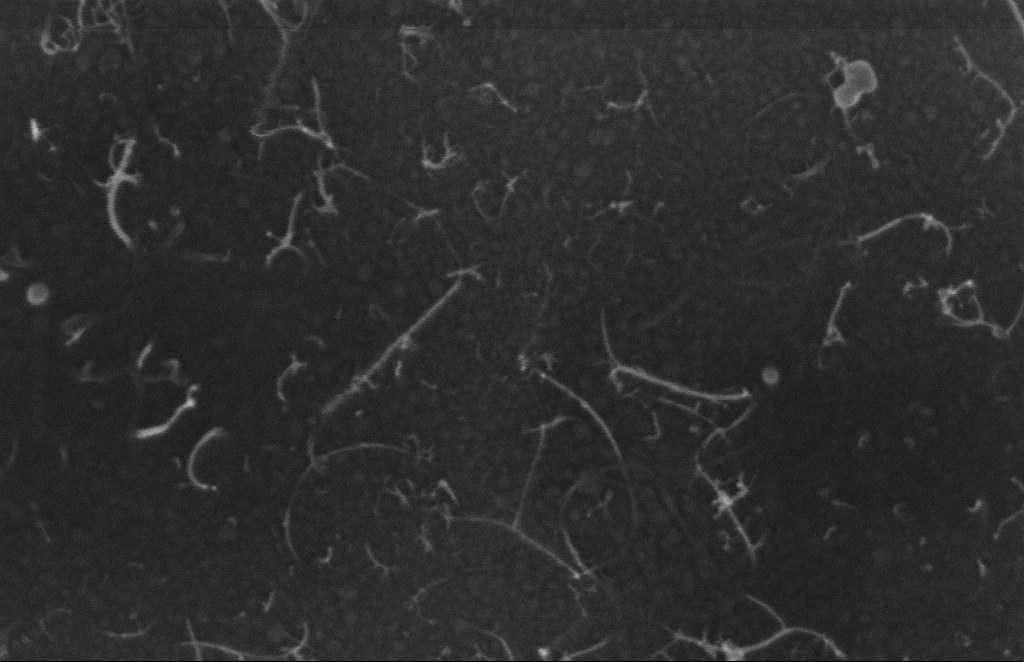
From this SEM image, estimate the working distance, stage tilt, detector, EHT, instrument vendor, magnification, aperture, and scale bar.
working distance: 8 mm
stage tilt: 0°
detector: InLens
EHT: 5 kV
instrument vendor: Zeiss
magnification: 270.4 K X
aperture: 20 µm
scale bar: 100 nm